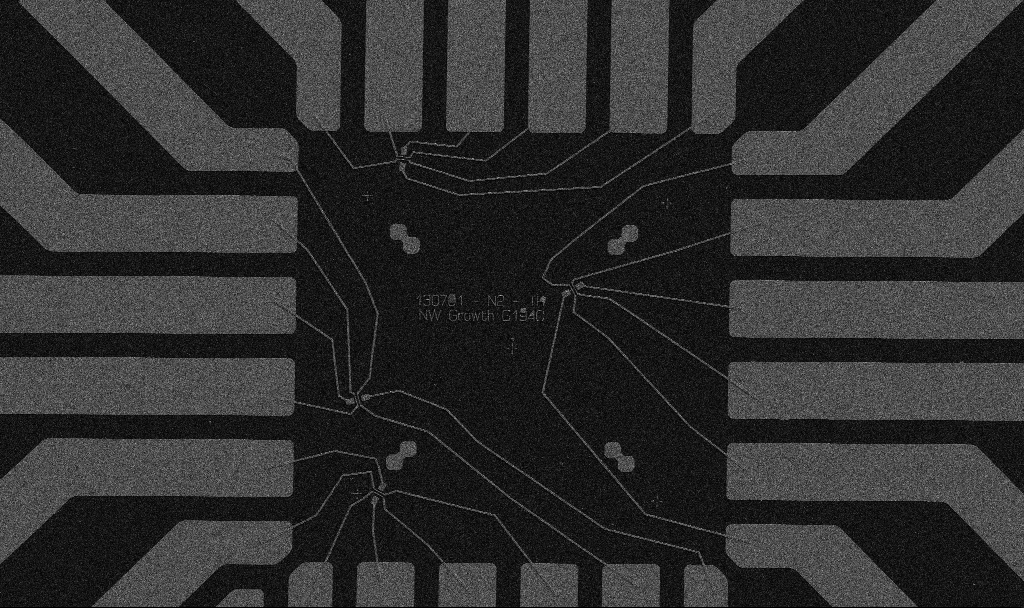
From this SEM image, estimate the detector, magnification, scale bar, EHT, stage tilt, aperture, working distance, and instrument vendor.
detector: SE2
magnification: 1 K X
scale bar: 20000 nm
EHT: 5 kV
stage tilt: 0°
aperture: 30 µm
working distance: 10.7 mm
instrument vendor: Zeiss